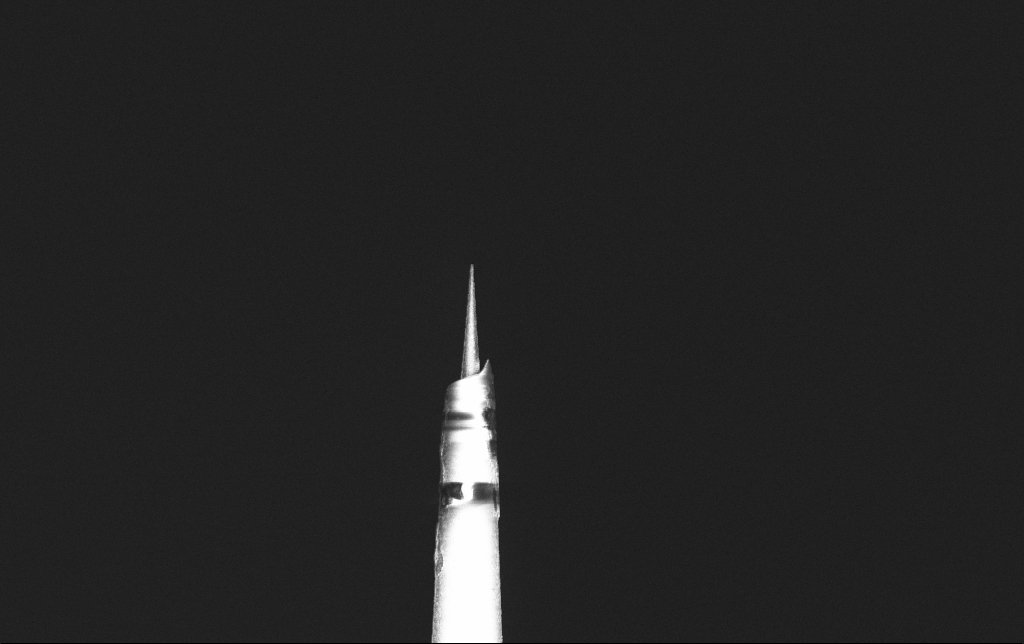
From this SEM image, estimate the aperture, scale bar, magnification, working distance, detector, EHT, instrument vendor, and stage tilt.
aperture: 30 µm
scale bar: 10000 nm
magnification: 5 K X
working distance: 6.5 mm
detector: InLens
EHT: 2 kV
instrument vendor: Zeiss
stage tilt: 0°